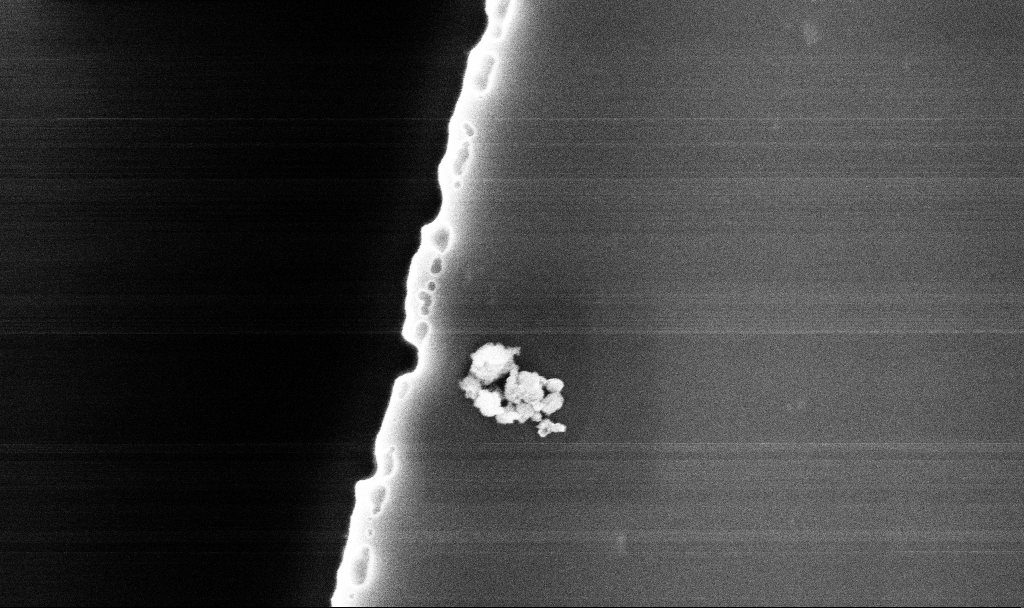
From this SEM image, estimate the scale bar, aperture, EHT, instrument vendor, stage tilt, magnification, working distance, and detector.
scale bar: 1000 nm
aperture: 30 µm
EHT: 5 kV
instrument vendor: Zeiss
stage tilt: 0°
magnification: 66.91 K X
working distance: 10.1 mm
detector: InLens